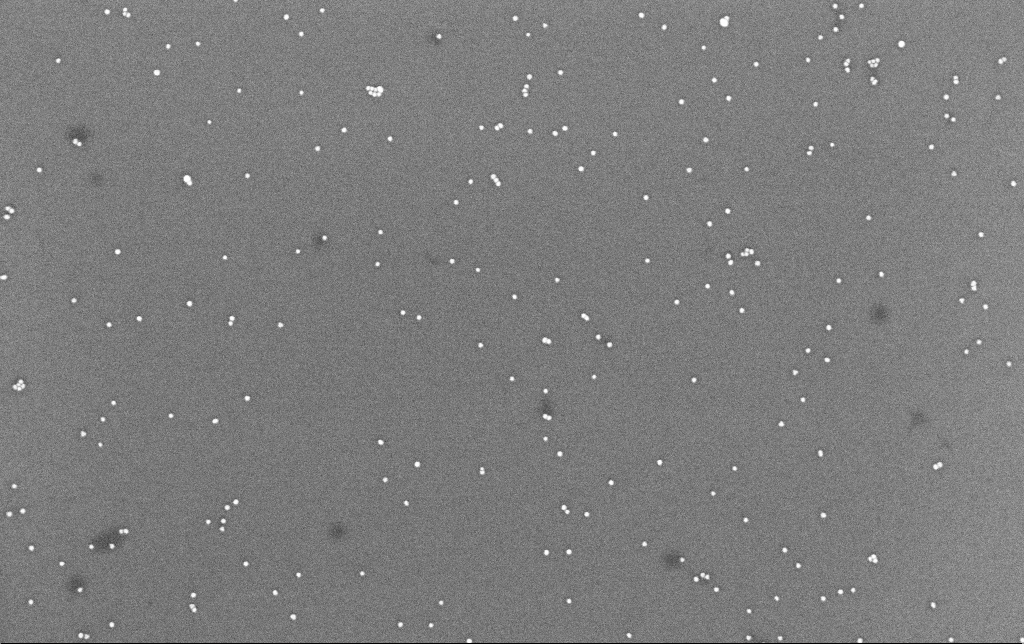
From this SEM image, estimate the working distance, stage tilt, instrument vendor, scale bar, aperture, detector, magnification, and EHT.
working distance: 6.6 mm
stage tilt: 0°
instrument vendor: Zeiss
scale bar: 200 nm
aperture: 30 µm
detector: InLens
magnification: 100 K X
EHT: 8 kV